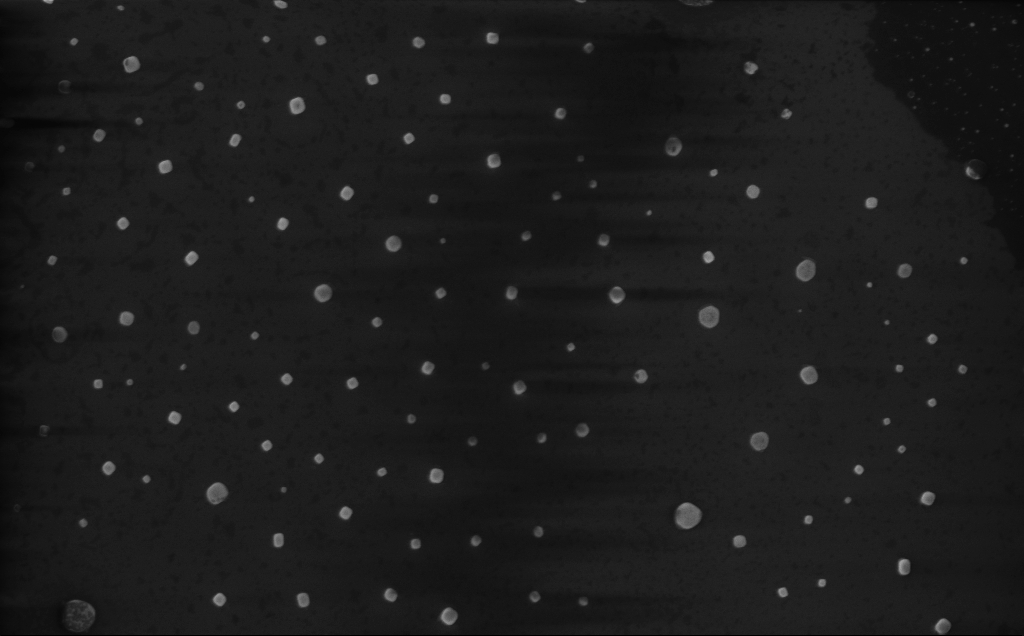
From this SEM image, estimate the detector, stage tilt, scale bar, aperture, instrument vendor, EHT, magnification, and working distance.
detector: InLens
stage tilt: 0°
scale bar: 1000 nm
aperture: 30 µm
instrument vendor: Zeiss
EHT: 10 kV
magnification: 26.67 K X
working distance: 5 mm